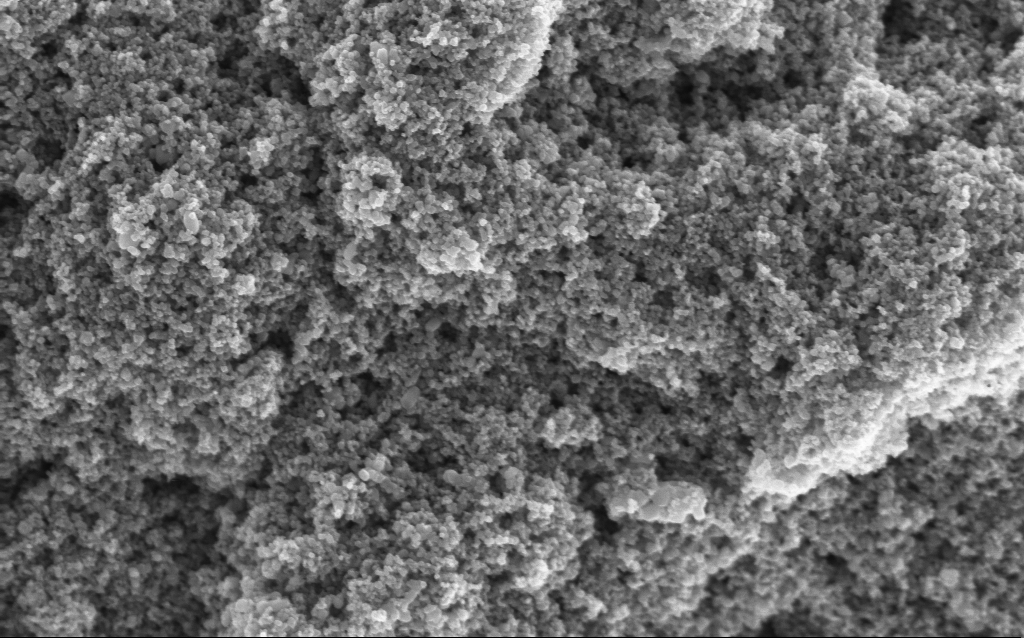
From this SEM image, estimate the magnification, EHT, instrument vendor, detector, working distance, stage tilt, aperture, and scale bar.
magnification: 68.59 K X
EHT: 5 kV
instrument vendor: Zeiss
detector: InLens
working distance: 4 mm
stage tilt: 0°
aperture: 30 µm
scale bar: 1000 nm